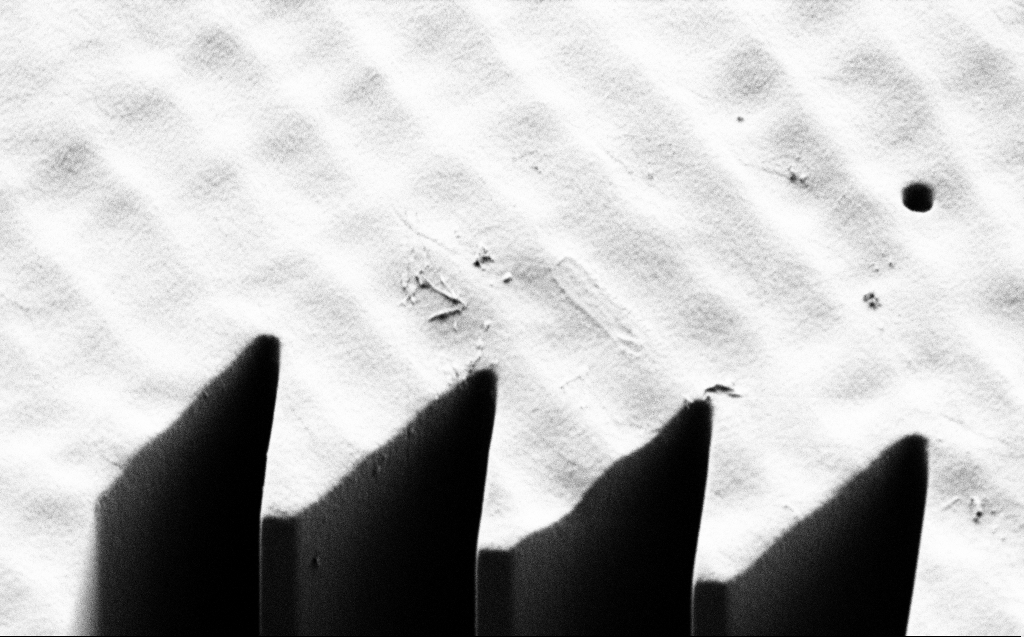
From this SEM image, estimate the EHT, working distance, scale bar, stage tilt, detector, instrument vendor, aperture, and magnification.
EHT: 1 kV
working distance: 7 mm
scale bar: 10000 nm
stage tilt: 45°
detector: SE2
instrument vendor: Zeiss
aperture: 30 µm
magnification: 6.97 K X